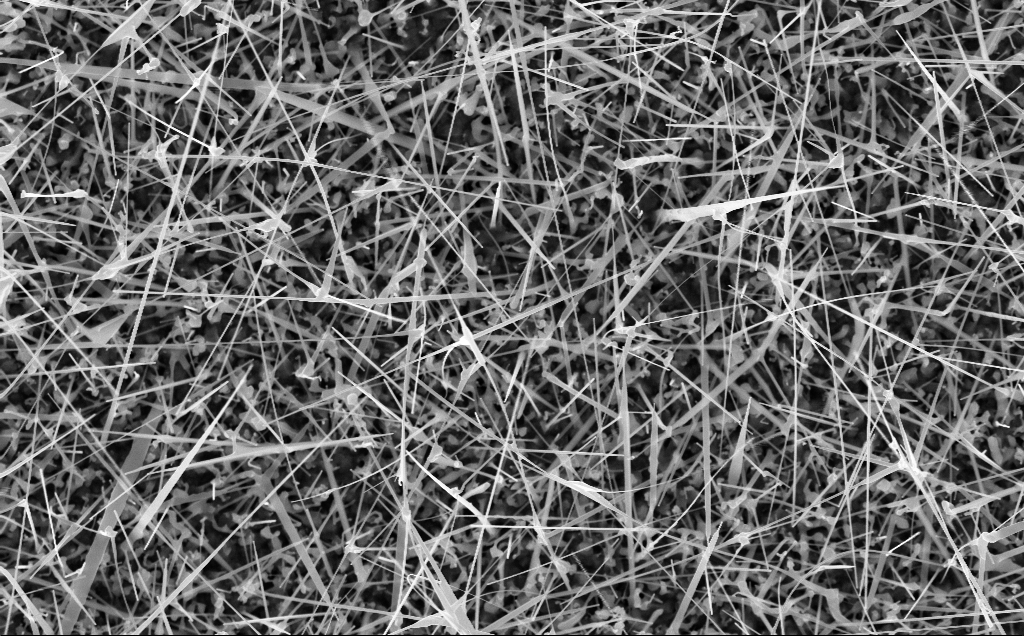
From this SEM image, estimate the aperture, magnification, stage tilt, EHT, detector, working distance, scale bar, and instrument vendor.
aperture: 30 µm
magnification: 21.32 K X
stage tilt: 0°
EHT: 10 kV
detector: InLens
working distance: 7 mm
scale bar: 2000 nm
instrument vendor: Zeiss